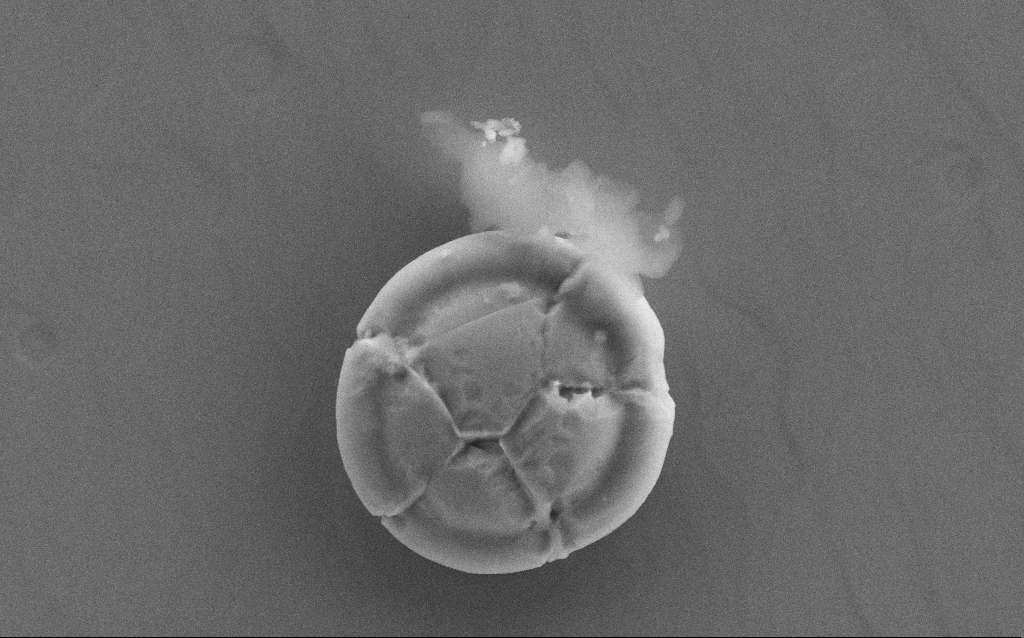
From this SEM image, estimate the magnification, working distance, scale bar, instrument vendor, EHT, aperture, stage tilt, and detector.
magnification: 30.82 K X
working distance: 2 mm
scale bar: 2000 nm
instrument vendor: Zeiss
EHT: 10 kV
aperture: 30 µm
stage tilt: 0°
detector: SE2